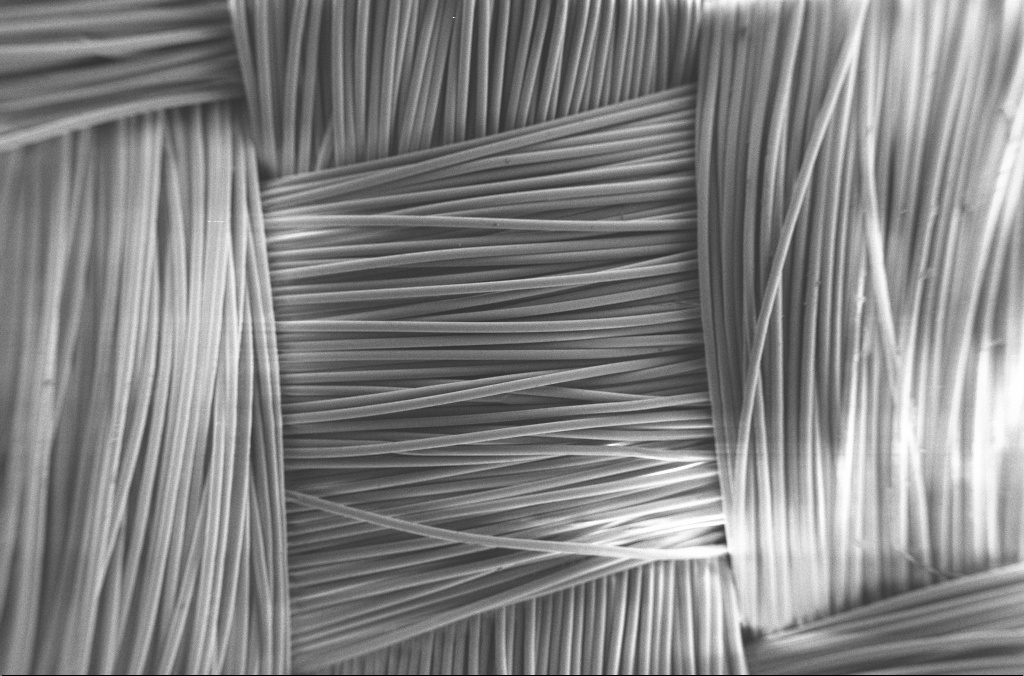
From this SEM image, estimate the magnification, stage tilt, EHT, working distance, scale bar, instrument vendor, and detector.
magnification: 0.2 K X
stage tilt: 0°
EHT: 1.5 kV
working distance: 4.6 mm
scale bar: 100000 nm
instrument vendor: Zeiss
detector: SE2